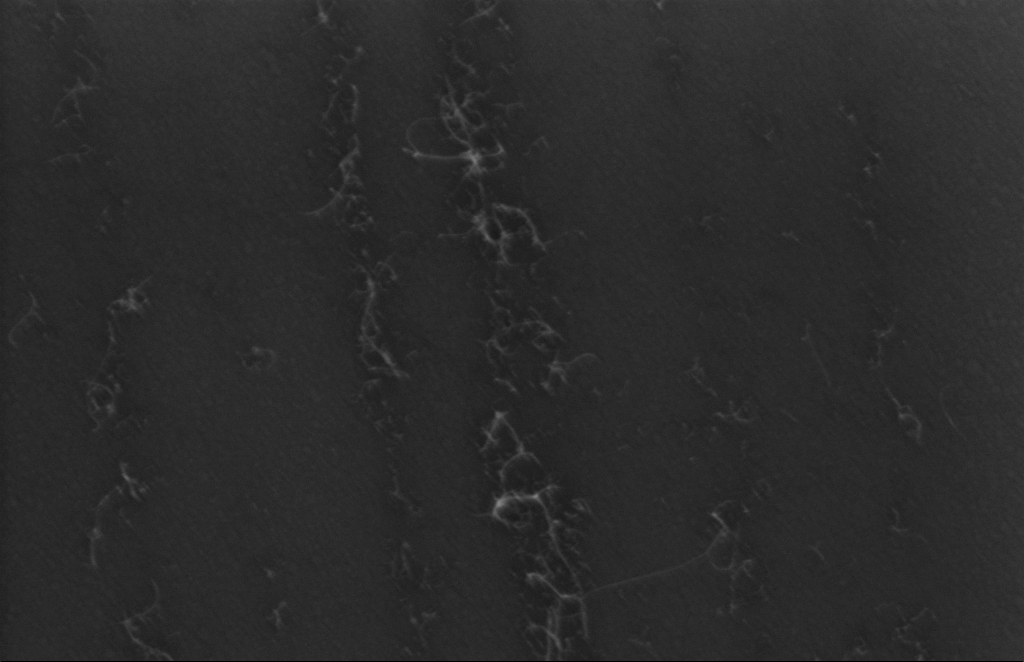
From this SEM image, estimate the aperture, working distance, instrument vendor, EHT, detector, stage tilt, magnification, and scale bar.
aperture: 20 µm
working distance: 5 mm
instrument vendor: Zeiss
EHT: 5 kV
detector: InLens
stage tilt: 0°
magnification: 183.19 K X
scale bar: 200 nm